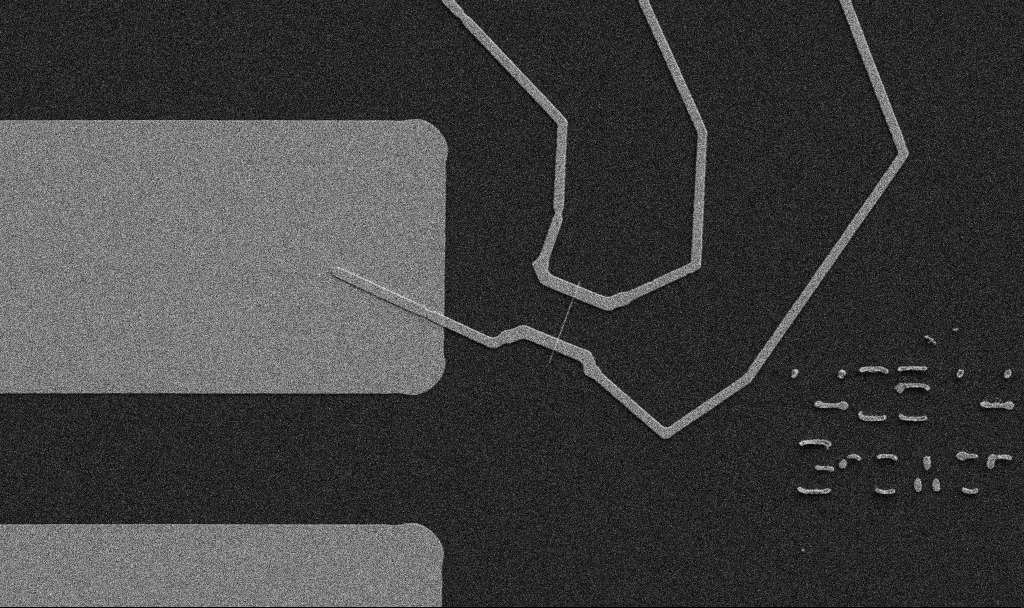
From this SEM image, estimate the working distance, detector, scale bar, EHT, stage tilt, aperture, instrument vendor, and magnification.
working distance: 10.7 mm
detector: SE2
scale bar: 10000 nm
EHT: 5 kV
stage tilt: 0°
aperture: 30 µm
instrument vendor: Zeiss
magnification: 5 K X